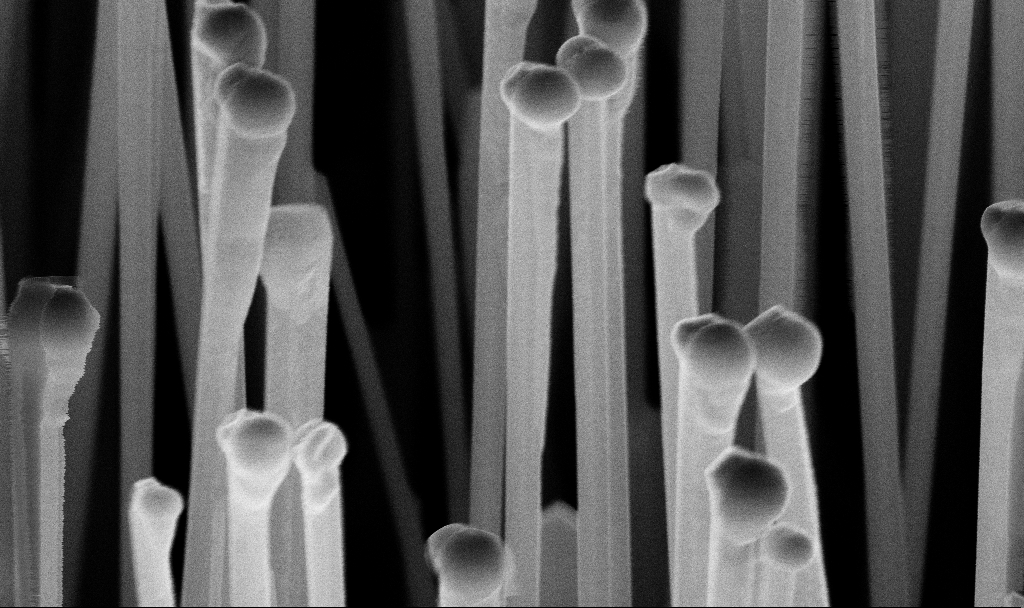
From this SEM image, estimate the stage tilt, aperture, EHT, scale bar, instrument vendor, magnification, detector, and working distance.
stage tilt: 45°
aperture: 30 µm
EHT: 10 kV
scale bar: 200 nm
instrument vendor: Zeiss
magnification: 124.06 K X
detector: InLens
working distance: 7.2 mm